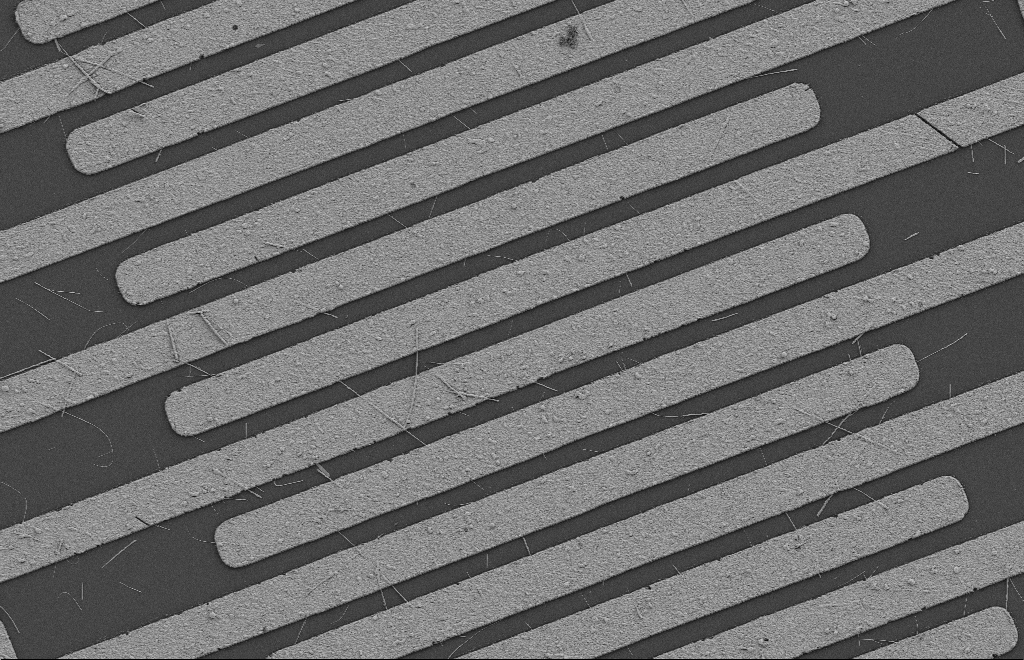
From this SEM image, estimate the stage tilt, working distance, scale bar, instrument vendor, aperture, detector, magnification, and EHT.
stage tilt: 0°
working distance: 8 mm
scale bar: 10000 nm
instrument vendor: Zeiss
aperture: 20 µm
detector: SE2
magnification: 4.28 K X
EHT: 2 kV